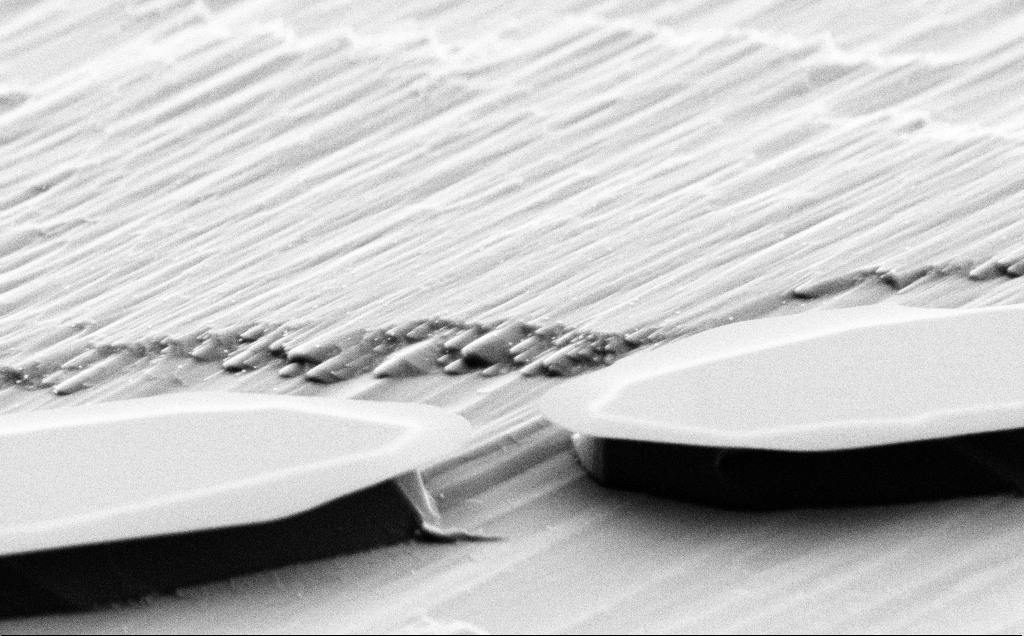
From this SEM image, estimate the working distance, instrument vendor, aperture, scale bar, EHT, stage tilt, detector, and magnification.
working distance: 7 mm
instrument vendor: Zeiss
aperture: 30 µm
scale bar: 2000 nm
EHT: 5 kV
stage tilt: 70°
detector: SE2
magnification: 12.86 K X